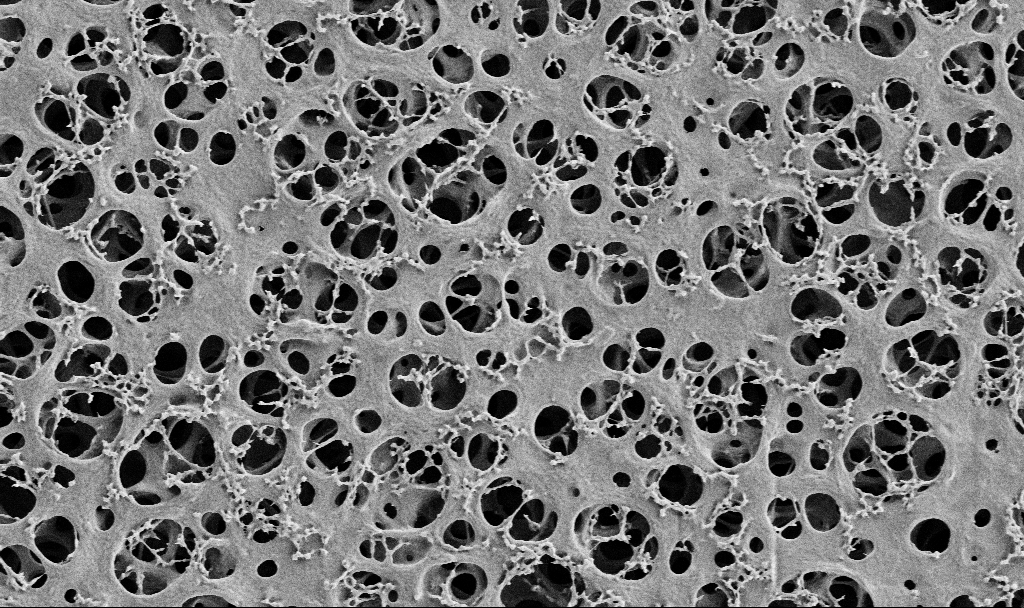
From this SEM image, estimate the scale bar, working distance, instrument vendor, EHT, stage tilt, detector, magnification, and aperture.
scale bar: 2000 nm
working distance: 3.5 mm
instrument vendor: Zeiss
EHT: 2 kV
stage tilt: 0°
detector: SE2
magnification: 10 K X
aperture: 30 µm